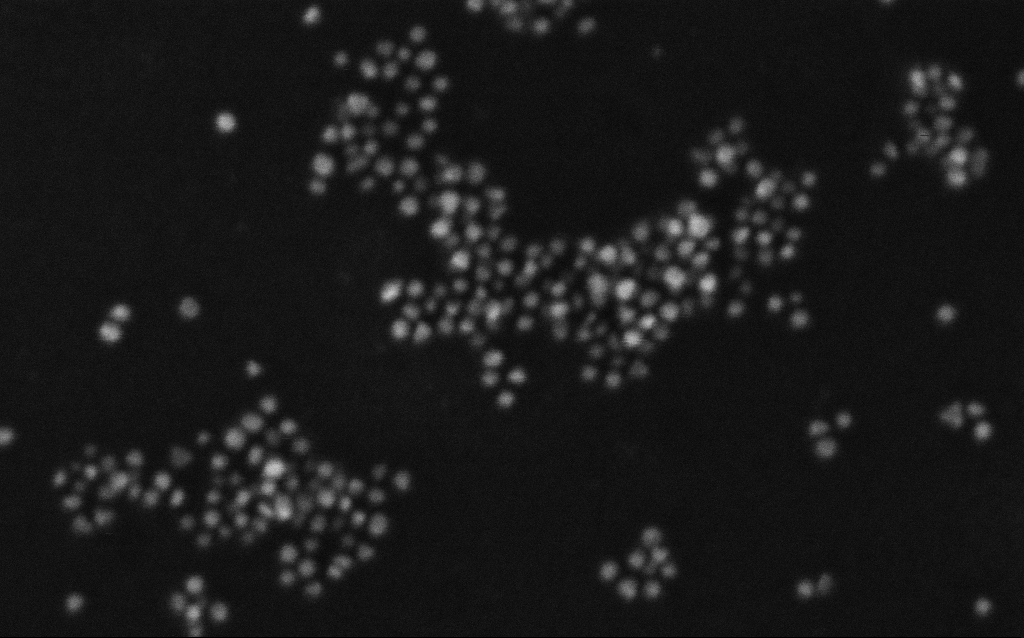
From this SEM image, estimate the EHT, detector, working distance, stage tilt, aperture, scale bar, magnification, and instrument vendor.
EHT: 10 kV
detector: InLens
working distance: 1.8 mm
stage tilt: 0°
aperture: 30 µm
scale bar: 100 nm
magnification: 443.82 K X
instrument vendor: Zeiss